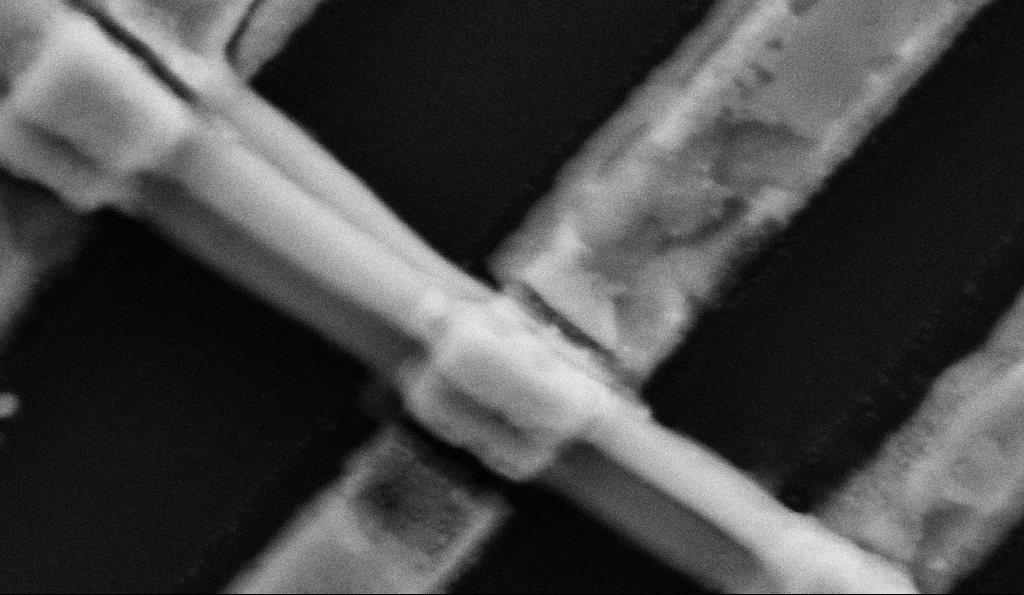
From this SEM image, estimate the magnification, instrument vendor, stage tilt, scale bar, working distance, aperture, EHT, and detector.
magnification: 228.15 K X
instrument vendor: Zeiss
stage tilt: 0°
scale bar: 200 nm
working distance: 8.5 mm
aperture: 30 µm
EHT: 5 kV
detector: SE2